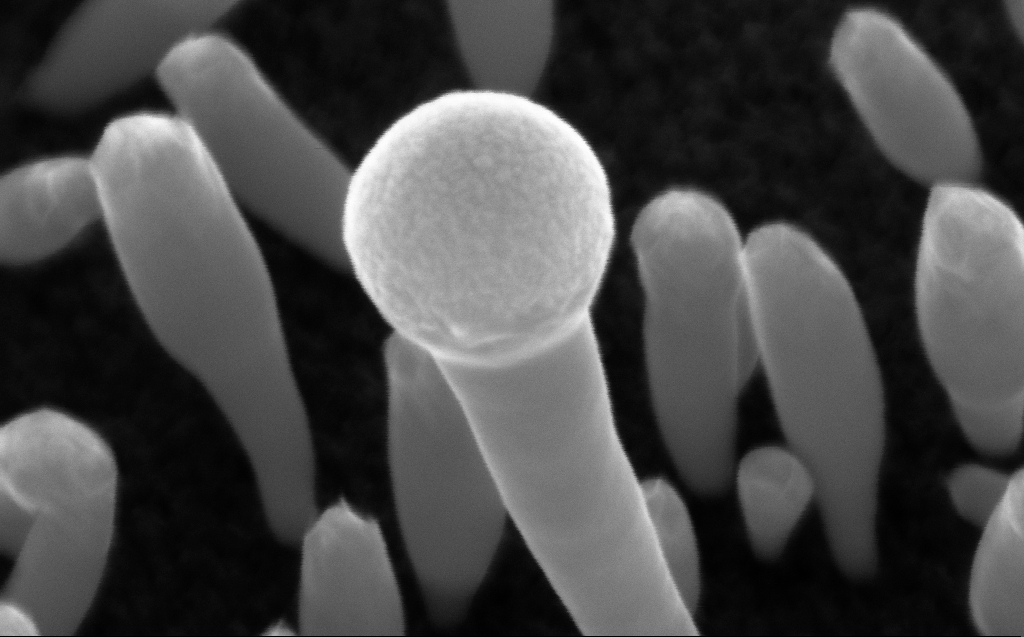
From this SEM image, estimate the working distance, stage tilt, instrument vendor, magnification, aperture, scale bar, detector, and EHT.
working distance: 5 mm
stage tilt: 45°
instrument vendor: Zeiss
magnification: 321.53 K X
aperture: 30 µm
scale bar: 200 nm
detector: InLens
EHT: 10 kV